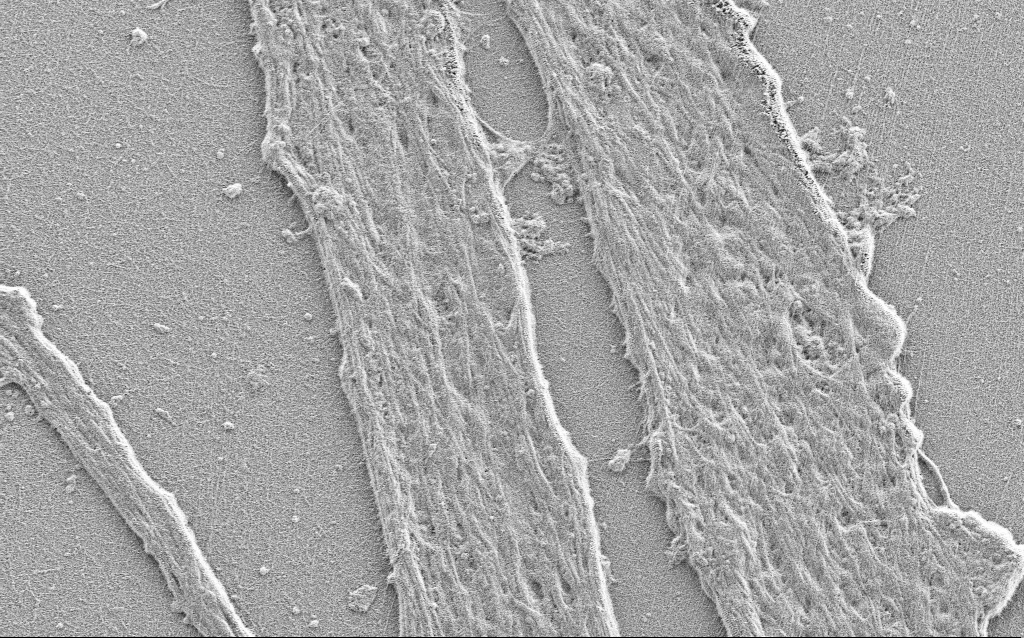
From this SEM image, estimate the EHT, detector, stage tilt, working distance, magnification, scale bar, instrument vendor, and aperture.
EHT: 0.9 kV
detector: SE2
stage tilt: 0°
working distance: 4 mm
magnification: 5 K X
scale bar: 10000 nm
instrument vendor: Zeiss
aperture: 30 µm